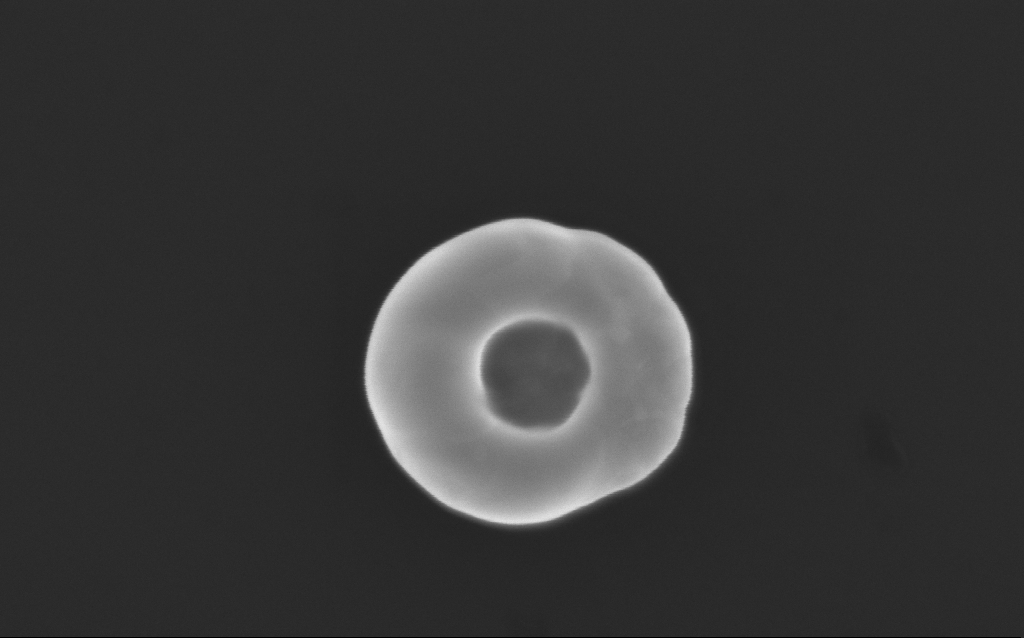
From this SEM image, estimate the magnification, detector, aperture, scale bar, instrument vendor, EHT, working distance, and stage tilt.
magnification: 154.31 K X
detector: InLens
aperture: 30 µm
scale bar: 200 nm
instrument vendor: Zeiss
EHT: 10 kV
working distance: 4 mm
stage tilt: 0°